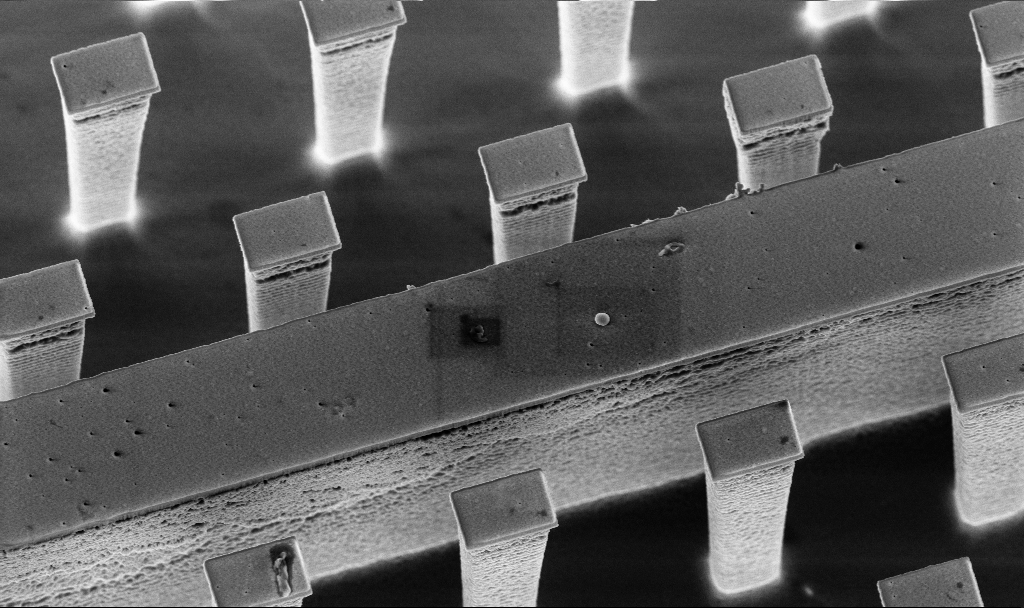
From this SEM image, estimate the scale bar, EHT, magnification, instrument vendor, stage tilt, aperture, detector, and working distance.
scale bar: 2000 nm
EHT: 5 kV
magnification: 7.89 K X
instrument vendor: Zeiss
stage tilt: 20°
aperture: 30 µm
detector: InLens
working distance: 3 mm